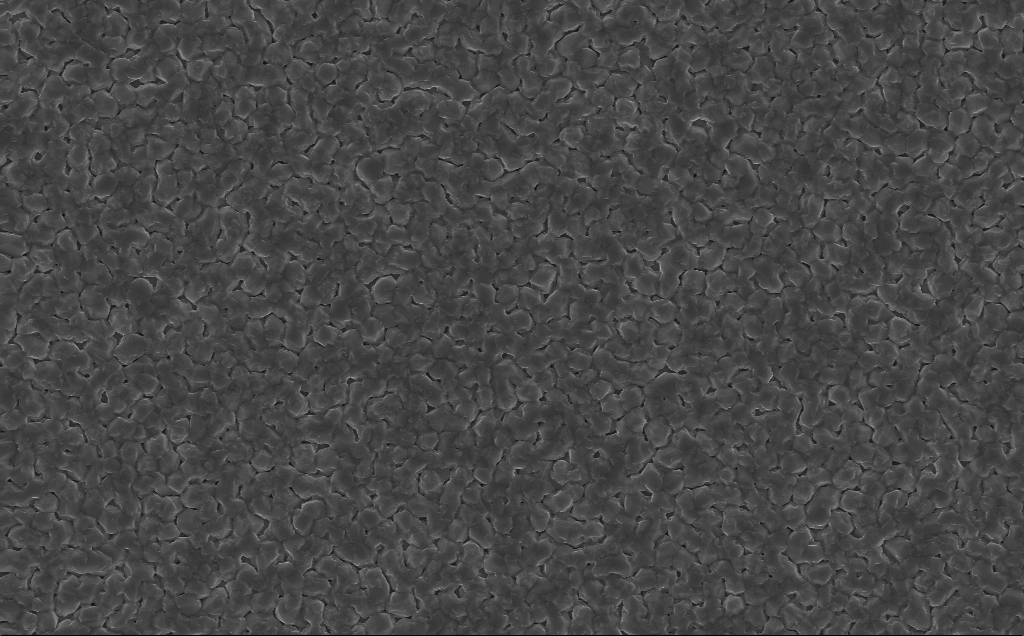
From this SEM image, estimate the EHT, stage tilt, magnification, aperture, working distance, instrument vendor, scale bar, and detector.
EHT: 10 kV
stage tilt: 0°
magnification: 9.02 K X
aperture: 30 µm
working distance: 8 mm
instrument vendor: Zeiss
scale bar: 2000 nm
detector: InLens